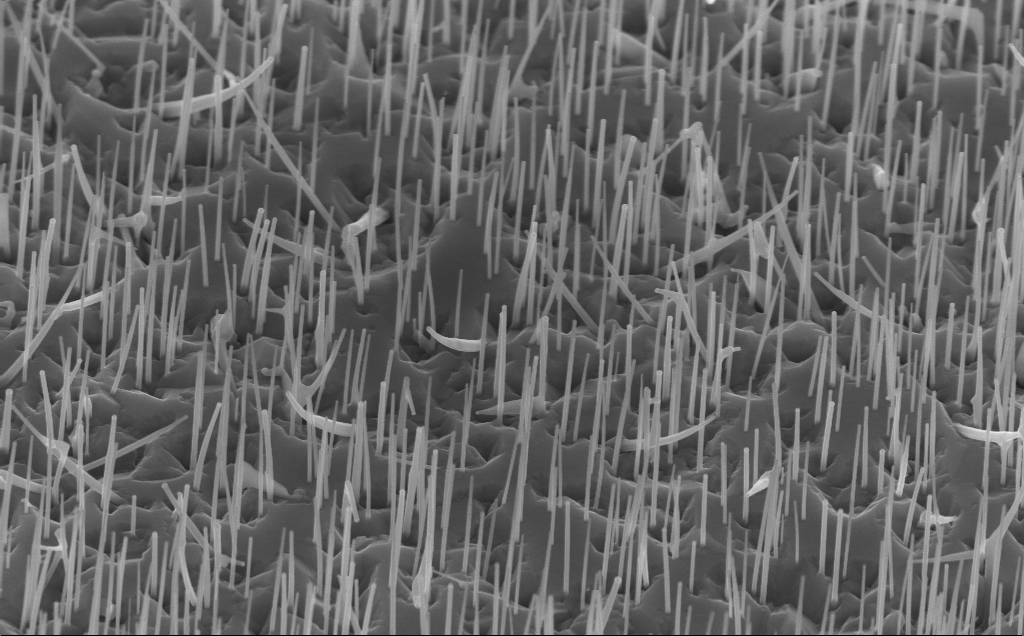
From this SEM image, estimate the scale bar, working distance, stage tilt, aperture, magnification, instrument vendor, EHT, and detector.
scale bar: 1000 nm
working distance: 5 mm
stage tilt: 45°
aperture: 30 µm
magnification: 40 K X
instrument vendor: Zeiss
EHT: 10 kV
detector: InLens